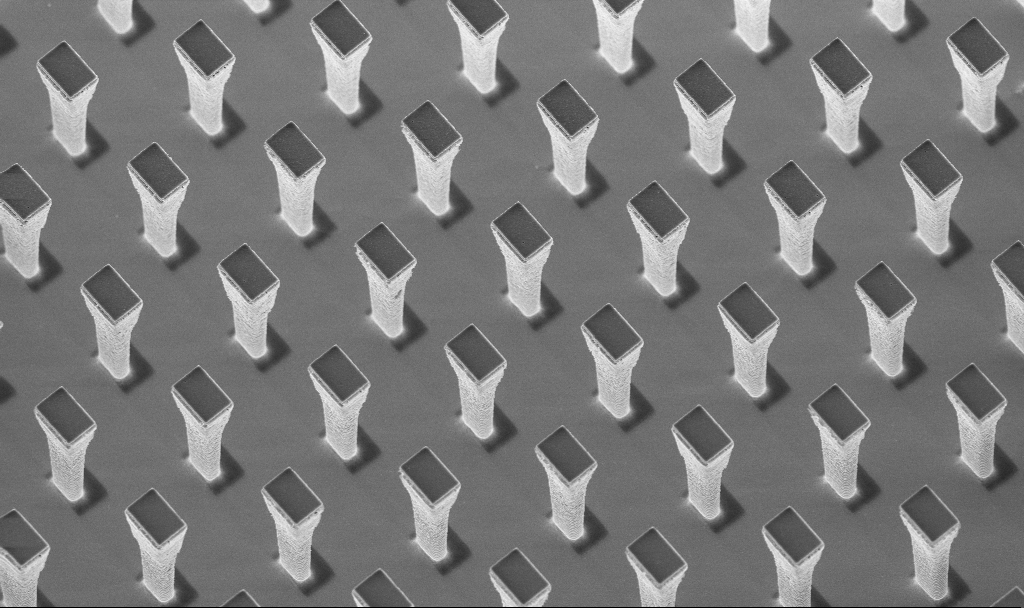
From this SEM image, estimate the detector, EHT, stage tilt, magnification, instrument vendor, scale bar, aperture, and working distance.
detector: InLens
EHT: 5 kV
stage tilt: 20°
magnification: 4.34 K X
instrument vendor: Zeiss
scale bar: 10000 nm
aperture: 30 µm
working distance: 4.3 mm